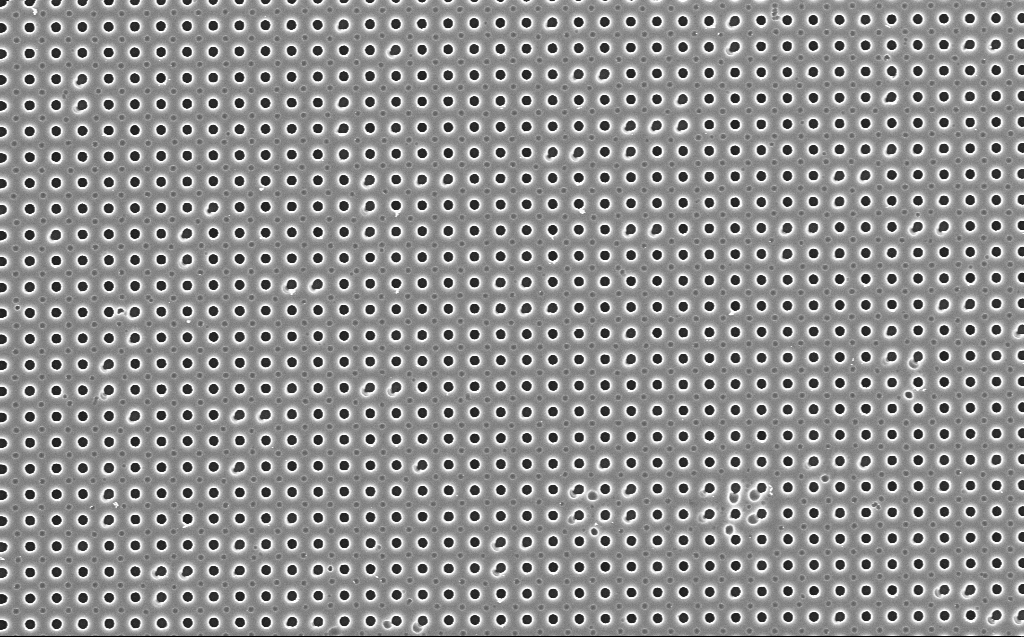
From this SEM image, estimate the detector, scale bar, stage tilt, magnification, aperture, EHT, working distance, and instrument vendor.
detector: InLens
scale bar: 2000 nm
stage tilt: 0°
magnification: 9.2 K X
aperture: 30 µm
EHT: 5 kV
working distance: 4 mm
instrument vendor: Zeiss